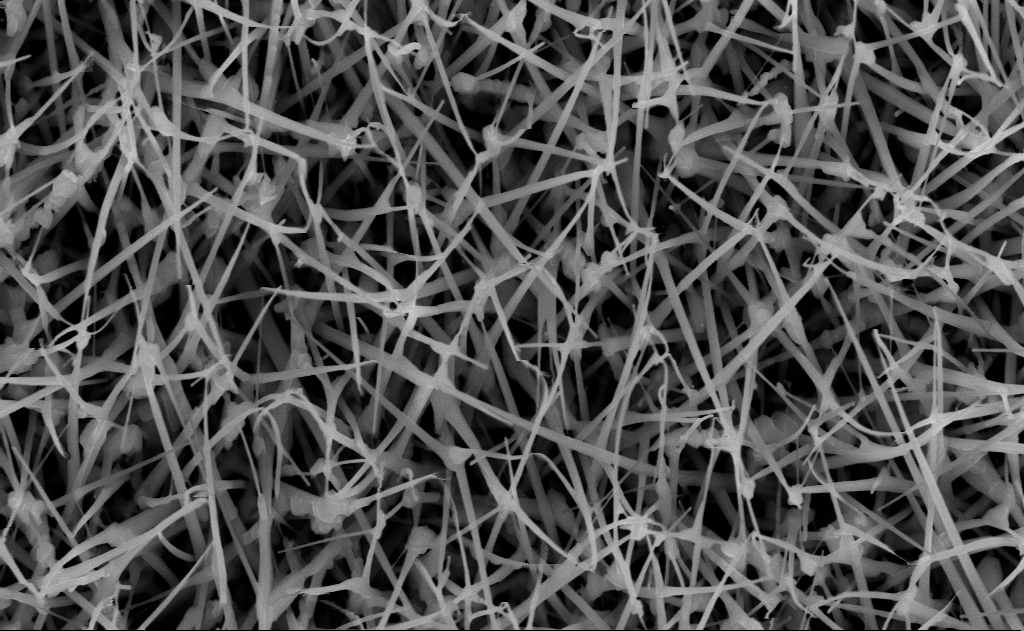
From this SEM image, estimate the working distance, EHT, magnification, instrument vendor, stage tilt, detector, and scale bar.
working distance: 10 mm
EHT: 10 kV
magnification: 40 K X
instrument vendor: Zeiss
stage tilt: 0°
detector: InLens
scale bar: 1000 nm